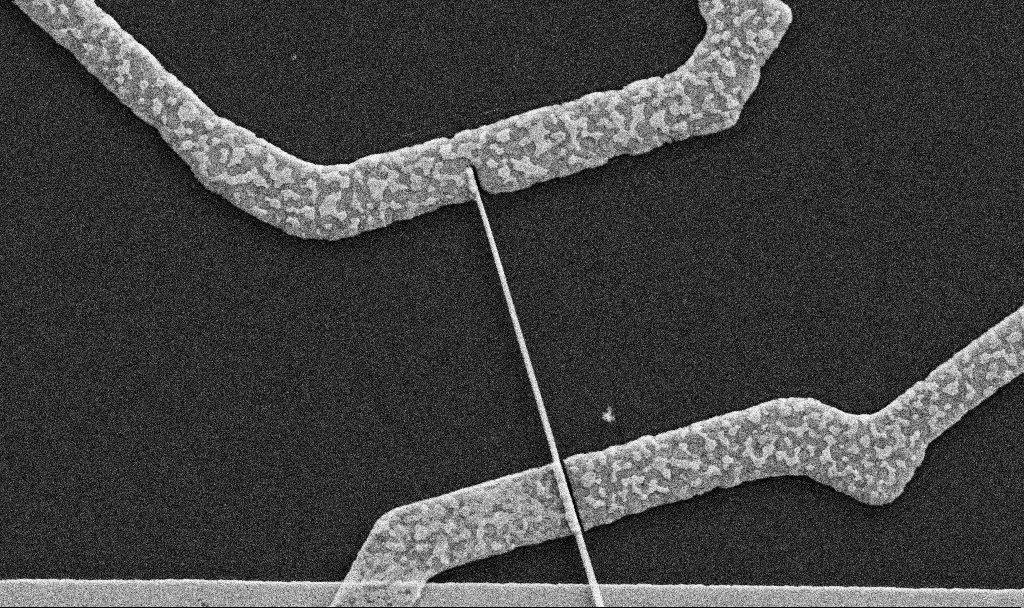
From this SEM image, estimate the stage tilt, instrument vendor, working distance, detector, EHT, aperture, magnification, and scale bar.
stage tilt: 0°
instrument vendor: Zeiss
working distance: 6.7 mm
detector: SE2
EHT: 5 kV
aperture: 30 µm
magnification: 30 K X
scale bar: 1000 nm